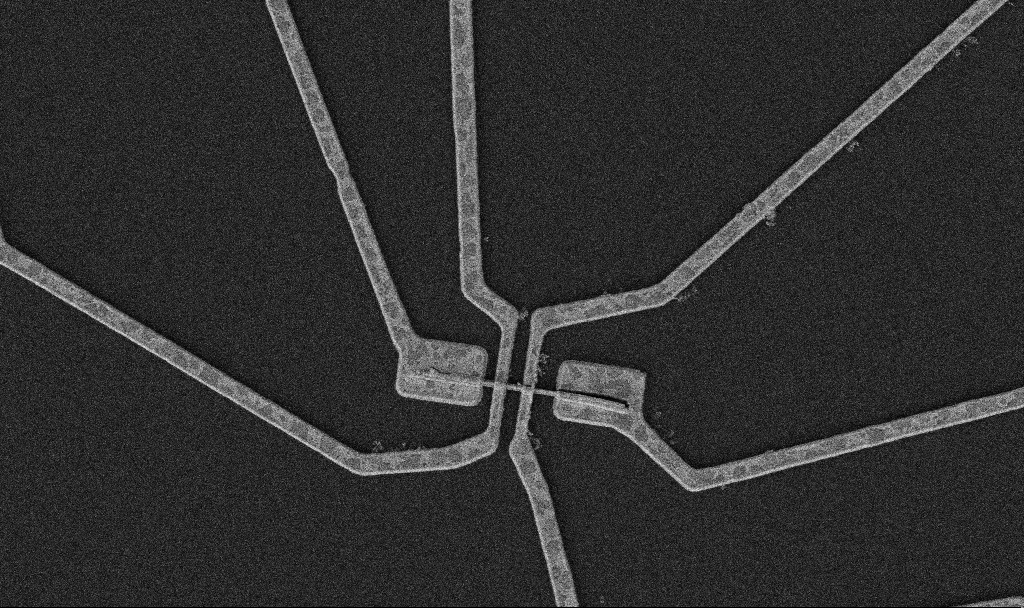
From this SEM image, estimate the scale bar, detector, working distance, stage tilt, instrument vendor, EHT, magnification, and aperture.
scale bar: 2000 nm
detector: SE2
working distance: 10.7 mm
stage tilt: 0°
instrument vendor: Zeiss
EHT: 5 kV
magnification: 10 K X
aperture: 30 µm